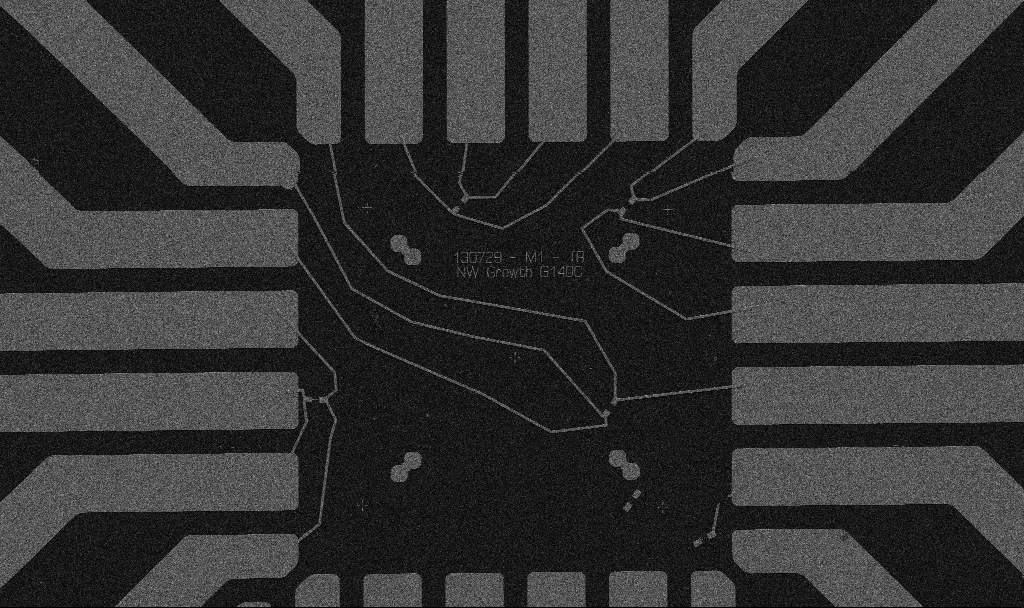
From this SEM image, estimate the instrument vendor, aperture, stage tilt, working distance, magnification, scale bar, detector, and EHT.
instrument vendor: Zeiss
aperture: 30 µm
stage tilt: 0°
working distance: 10.7 mm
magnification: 1 K X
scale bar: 20000 nm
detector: SE2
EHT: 5 kV